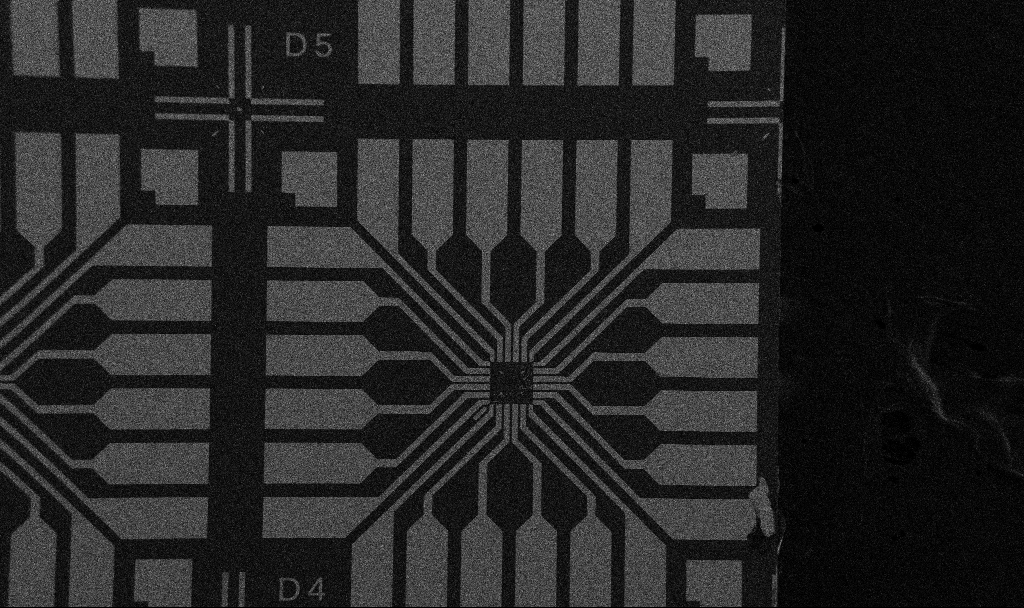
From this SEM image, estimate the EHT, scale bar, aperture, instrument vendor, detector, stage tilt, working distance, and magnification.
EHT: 5 kV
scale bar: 200000 nm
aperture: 30 µm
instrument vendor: Zeiss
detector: SE2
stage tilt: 0°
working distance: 10.7 mm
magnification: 0.1 K X